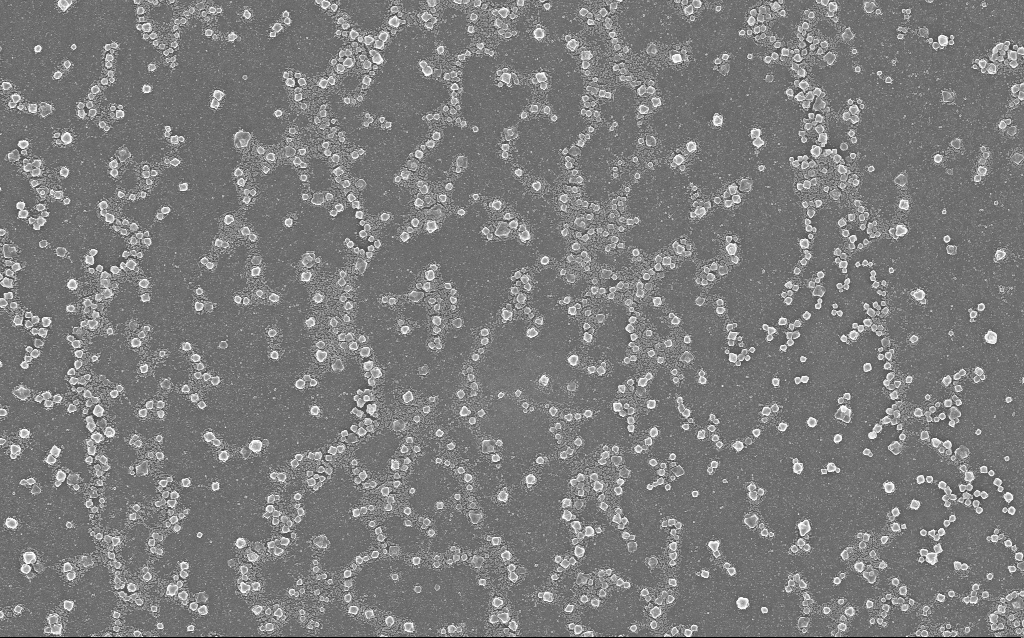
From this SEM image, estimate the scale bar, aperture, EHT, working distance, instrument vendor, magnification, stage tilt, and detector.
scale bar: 2000 nm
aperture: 30 µm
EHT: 20 kV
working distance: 1.5 mm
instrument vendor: Zeiss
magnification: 10 K X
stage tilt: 0°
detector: InLens